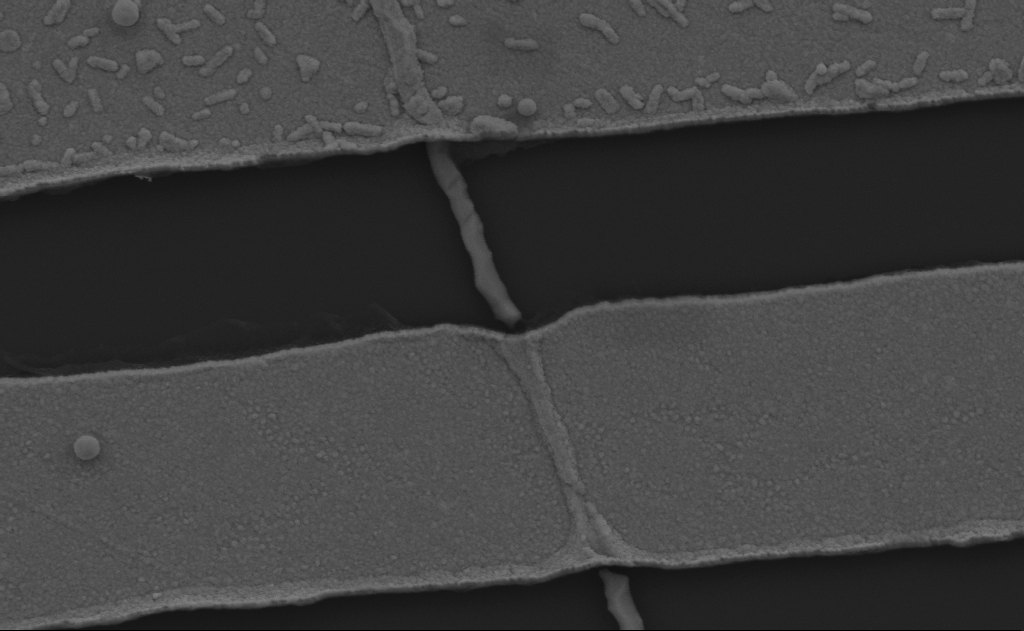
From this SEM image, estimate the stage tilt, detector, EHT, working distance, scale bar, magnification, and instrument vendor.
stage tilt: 0°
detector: SE2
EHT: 5 kV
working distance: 13 mm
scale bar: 1000 nm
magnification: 41.47 K X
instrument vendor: Zeiss